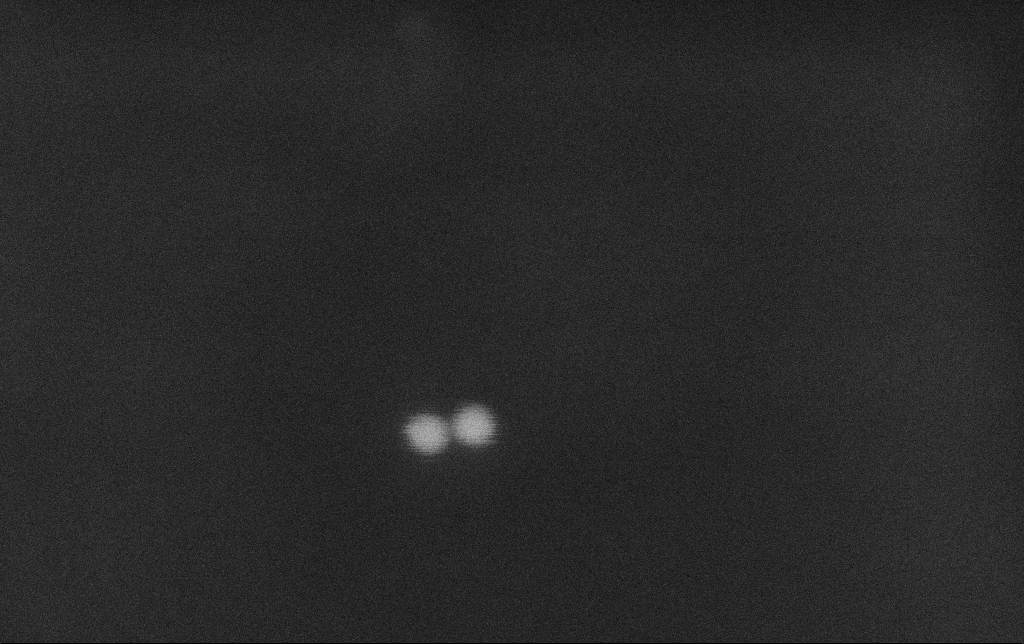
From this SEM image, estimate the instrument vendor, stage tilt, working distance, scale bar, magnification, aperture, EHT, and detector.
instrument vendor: Zeiss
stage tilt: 0°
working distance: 10.8 mm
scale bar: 20 nm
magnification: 1303.63 K X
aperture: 30 µm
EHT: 30 kV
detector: SE2